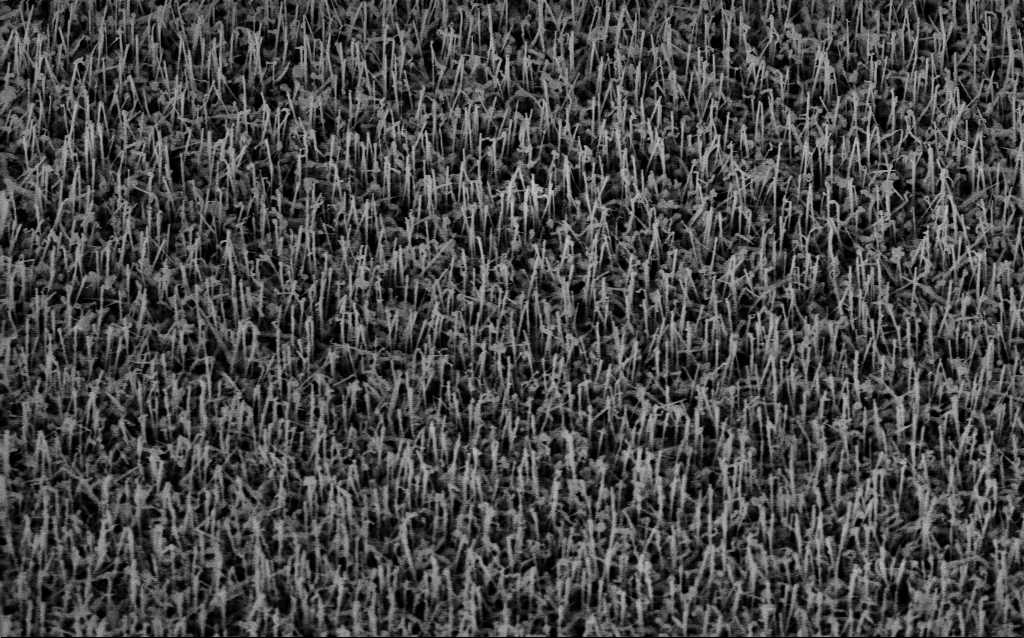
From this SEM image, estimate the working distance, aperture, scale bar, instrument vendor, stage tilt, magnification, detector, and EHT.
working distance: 7.3 mm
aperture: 30 µm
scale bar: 1000 nm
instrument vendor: Zeiss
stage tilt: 35°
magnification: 27.14 K X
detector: InLens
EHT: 10 kV